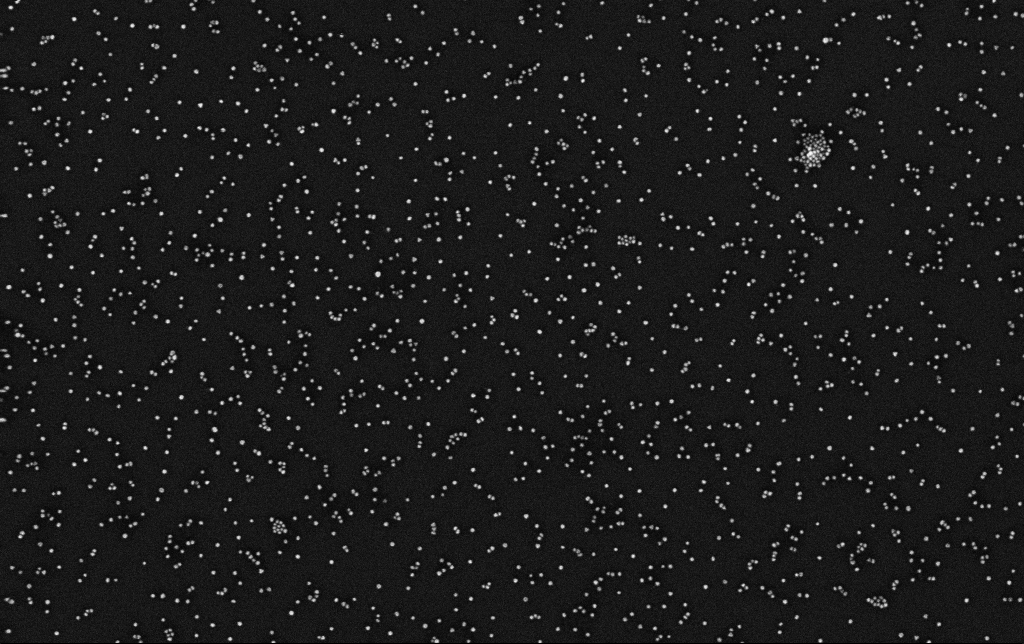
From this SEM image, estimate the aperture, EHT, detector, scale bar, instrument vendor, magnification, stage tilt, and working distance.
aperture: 30 µm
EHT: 10 kV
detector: InLens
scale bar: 200 nm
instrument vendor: Zeiss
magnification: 100 K X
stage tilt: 0°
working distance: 3.3 mm